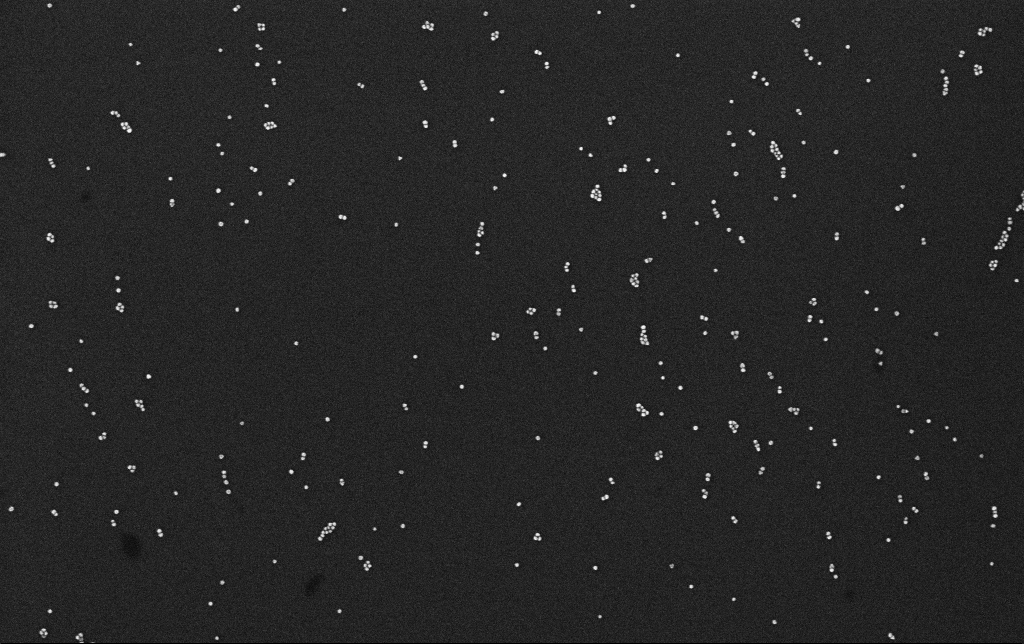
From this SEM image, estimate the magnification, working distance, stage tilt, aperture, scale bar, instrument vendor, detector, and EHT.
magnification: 100 K X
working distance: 3.1 mm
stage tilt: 0°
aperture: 30 µm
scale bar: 200 nm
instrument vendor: Zeiss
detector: InLens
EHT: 10 kV